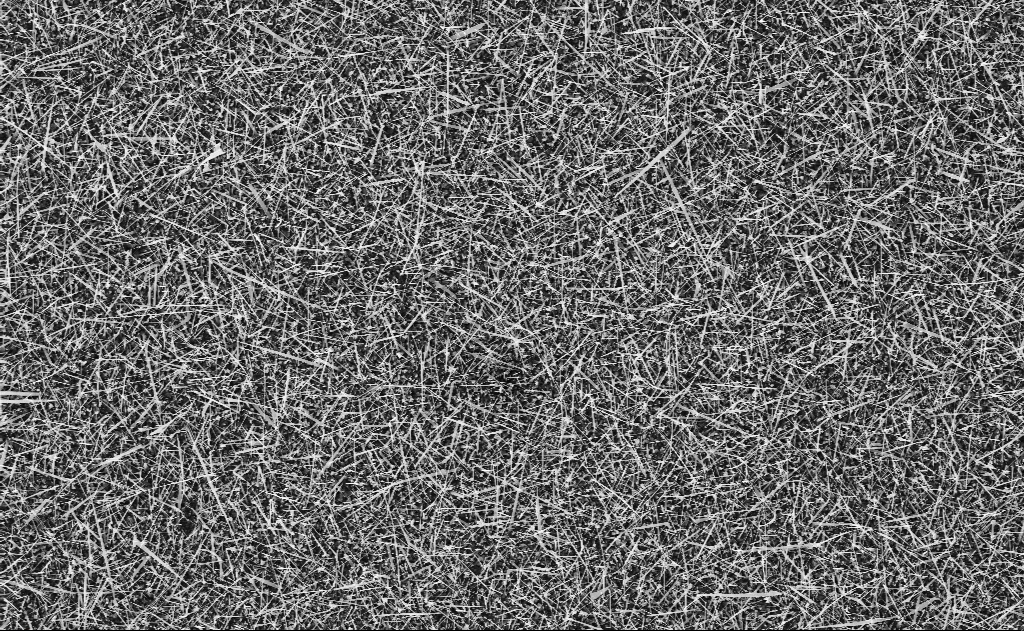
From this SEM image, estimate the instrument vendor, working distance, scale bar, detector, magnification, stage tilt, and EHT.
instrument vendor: Zeiss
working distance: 11 mm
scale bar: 10000 nm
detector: InLens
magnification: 5 K X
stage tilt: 0°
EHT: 10 kV